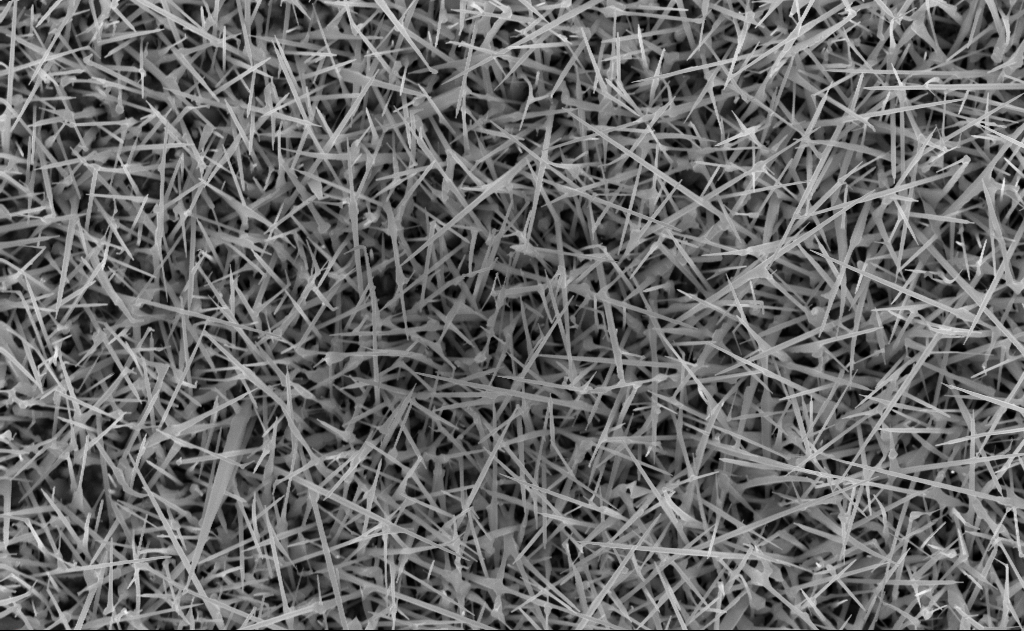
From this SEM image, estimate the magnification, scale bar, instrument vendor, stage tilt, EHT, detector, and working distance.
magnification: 20 K X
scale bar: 2000 nm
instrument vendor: Zeiss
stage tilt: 0°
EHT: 10 kV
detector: InLens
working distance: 10 mm